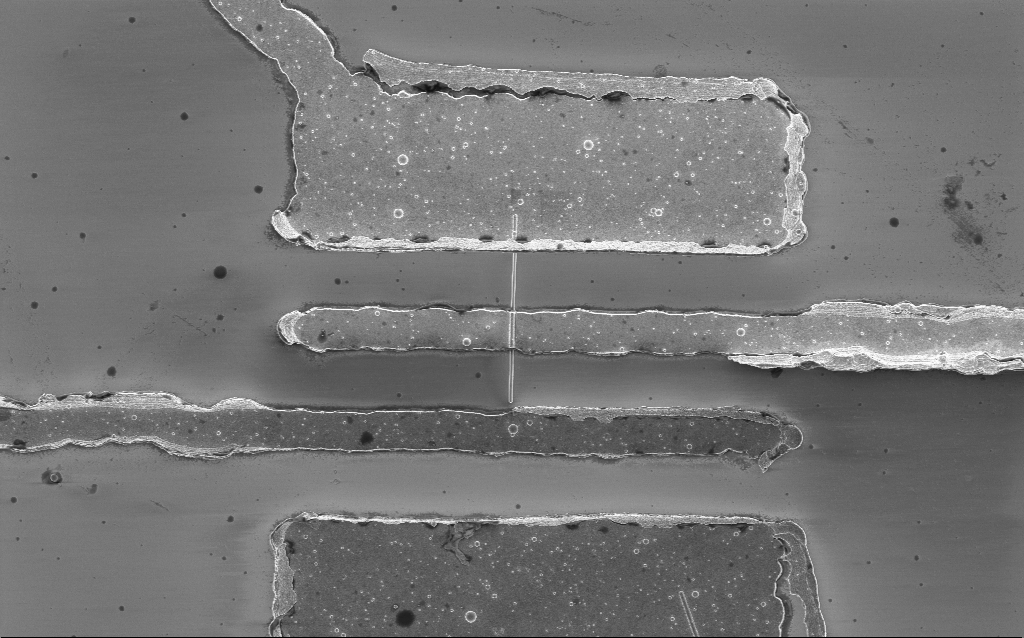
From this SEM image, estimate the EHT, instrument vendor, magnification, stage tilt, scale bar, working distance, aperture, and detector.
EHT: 2 kV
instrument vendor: Zeiss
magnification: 6.3 K X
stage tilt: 0°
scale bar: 10000 nm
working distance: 7.6 mm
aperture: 30 µm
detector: InLens